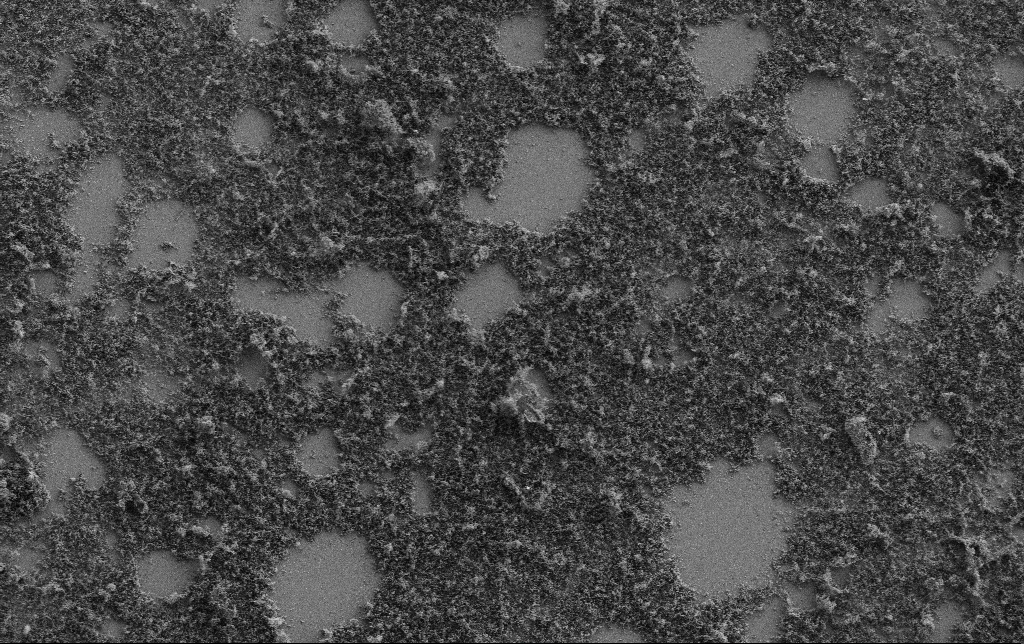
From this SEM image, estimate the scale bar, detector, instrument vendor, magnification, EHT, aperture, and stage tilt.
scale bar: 20000 nm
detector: SE2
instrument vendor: Zeiss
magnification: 1 K X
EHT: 2 kV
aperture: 30 µm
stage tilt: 0°